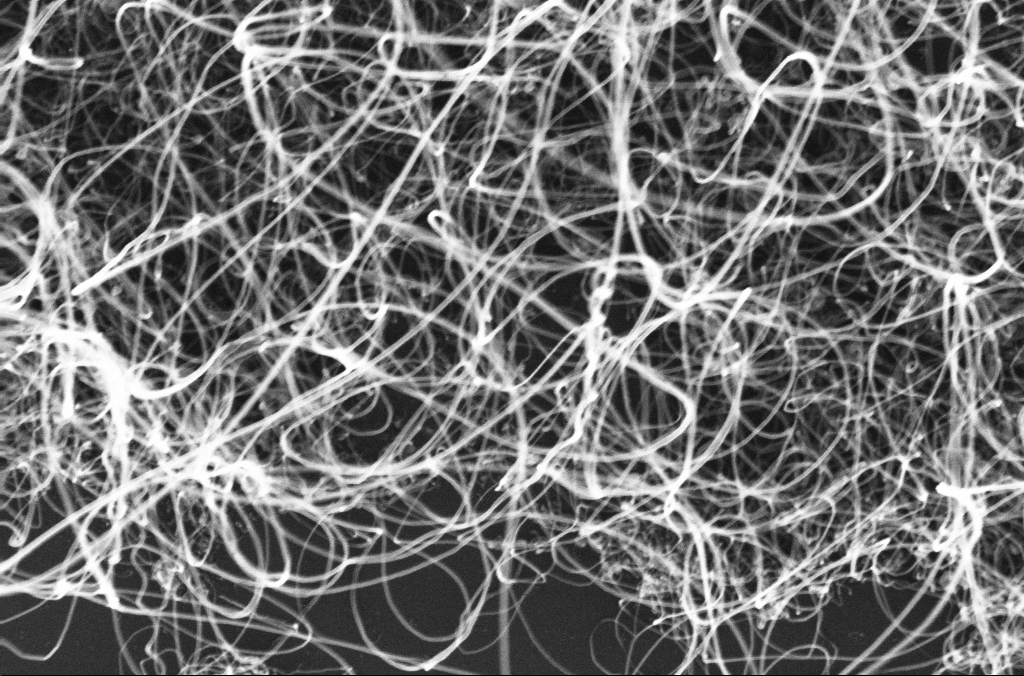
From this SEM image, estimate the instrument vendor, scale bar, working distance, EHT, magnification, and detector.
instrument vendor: Zeiss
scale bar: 200 nm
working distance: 3.3 mm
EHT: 10 kV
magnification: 99.65 K X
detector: InLens